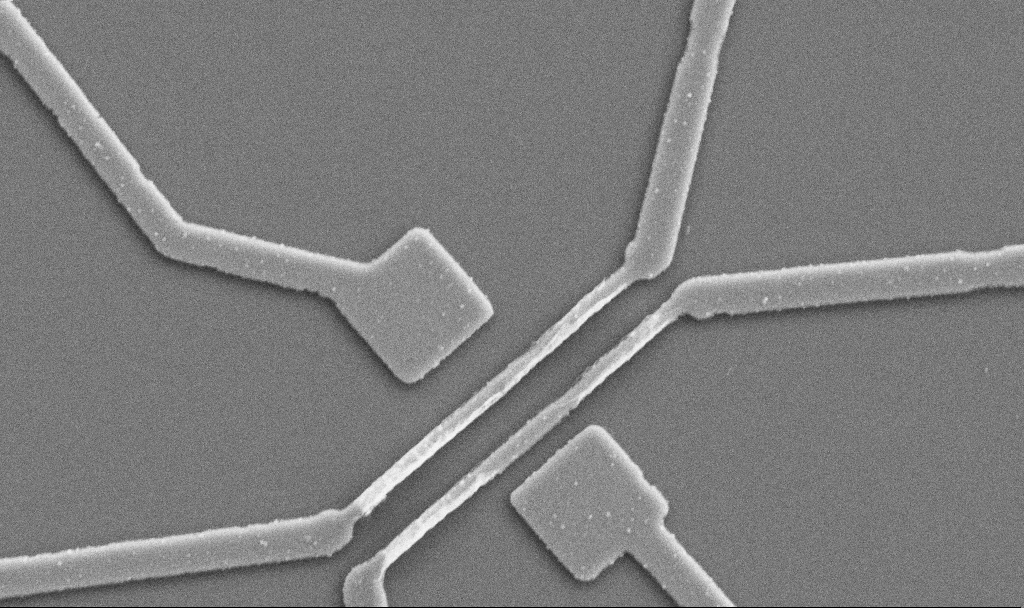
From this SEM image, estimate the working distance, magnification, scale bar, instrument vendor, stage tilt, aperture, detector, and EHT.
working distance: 10.7 mm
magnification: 20 K X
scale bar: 1000 nm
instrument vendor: Zeiss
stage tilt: -0°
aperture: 30 µm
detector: SE2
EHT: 5 kV